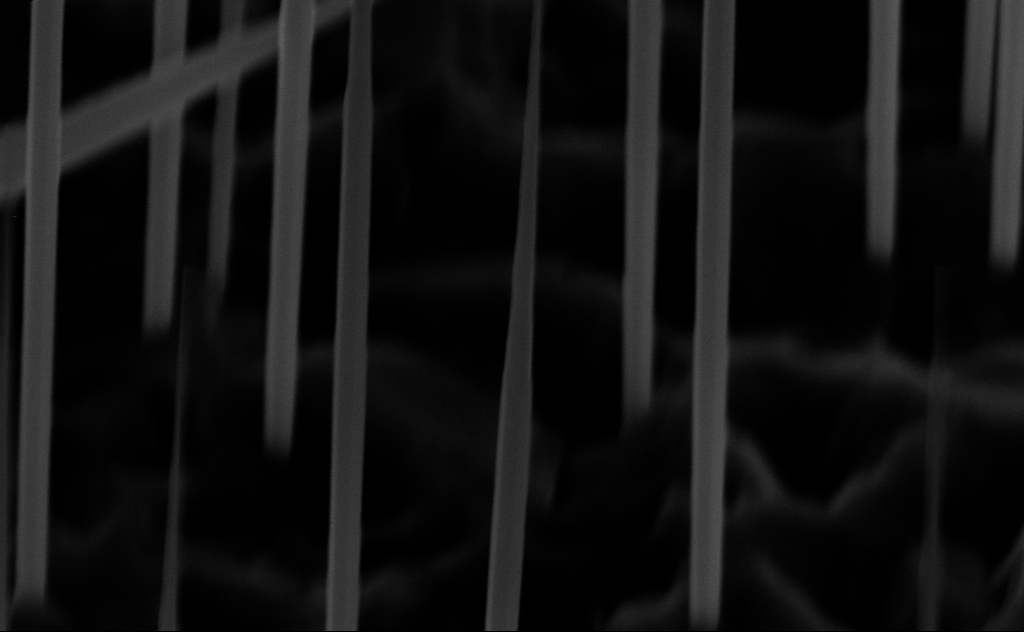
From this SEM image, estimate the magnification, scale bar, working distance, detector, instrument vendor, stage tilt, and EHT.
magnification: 80 K X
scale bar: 200 nm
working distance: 7 mm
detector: InLens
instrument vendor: Zeiss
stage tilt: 45°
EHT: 10 kV